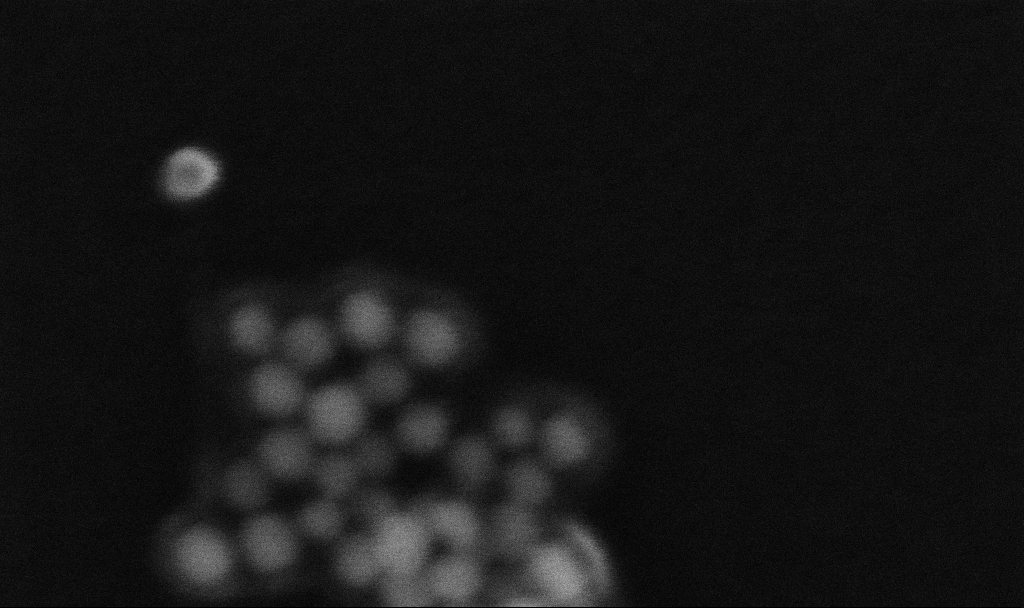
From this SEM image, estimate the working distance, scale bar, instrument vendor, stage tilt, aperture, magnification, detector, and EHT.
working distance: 3.3 mm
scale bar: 20 nm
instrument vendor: Zeiss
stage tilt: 0°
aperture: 30 µm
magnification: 1000 K X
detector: InLens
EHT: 10 kV